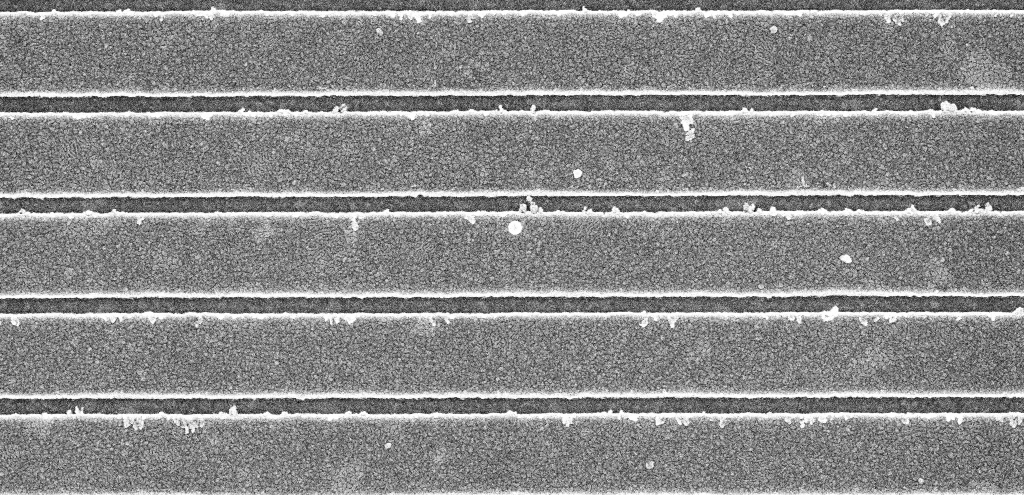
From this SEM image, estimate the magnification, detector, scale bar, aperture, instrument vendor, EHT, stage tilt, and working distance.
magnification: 53.05 K X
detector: InLens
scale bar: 1000 nm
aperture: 30 µm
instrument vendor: Zeiss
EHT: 5 kV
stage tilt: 0°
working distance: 5.3 mm